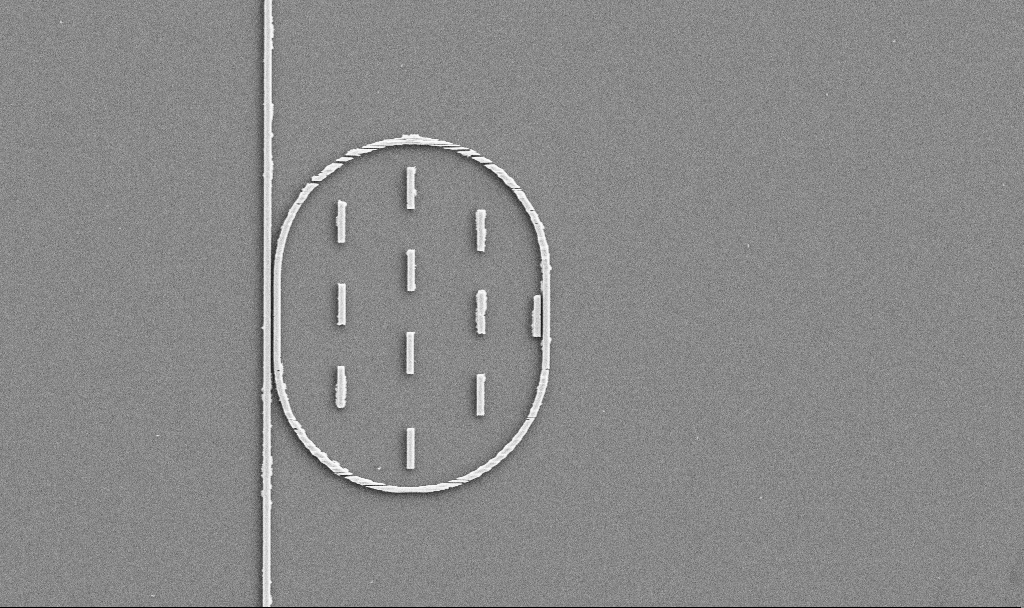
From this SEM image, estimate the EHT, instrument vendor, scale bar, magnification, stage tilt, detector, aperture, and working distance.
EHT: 5 kV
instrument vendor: Zeiss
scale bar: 10000 nm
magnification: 5.08 K X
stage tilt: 0°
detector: SE2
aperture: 30 µm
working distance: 7.5 mm